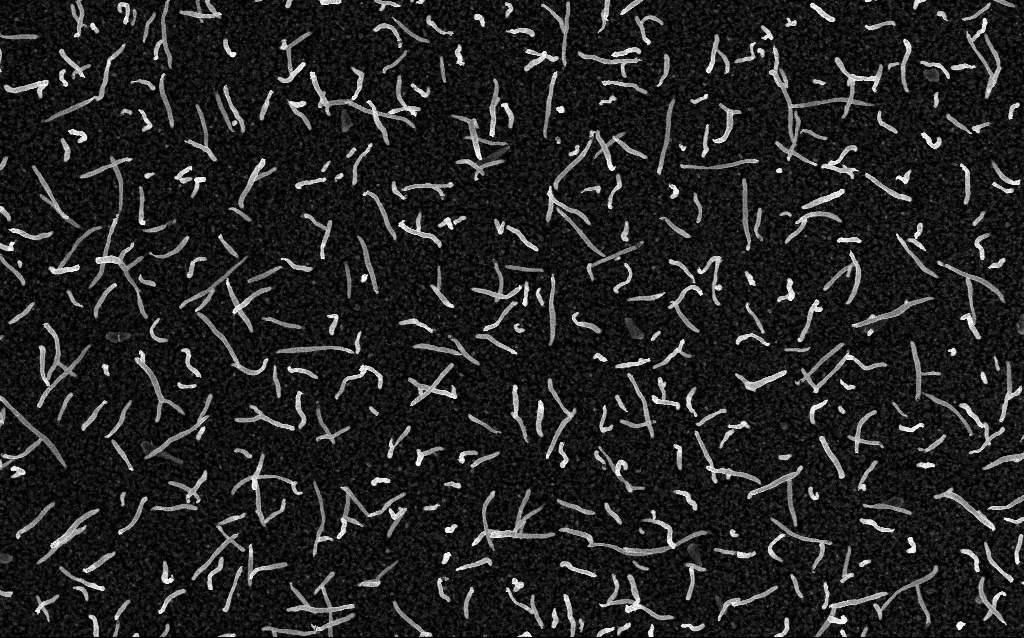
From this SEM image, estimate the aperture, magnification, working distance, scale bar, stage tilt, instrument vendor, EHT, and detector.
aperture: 30 µm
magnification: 20 K X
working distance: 2 mm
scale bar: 1000 nm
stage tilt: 0°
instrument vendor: Zeiss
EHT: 5 kV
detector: InLens